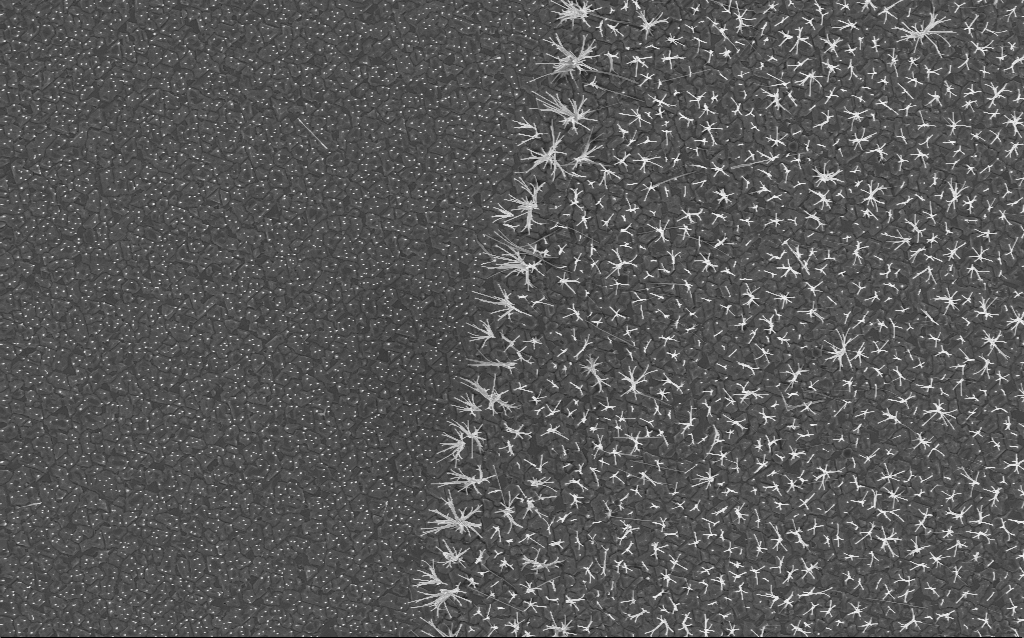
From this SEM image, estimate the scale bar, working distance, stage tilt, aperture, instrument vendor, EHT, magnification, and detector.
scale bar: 10000 nm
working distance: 6.7 mm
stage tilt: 0°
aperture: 30 µm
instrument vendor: Zeiss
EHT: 5 kV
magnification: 5 K X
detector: InLens